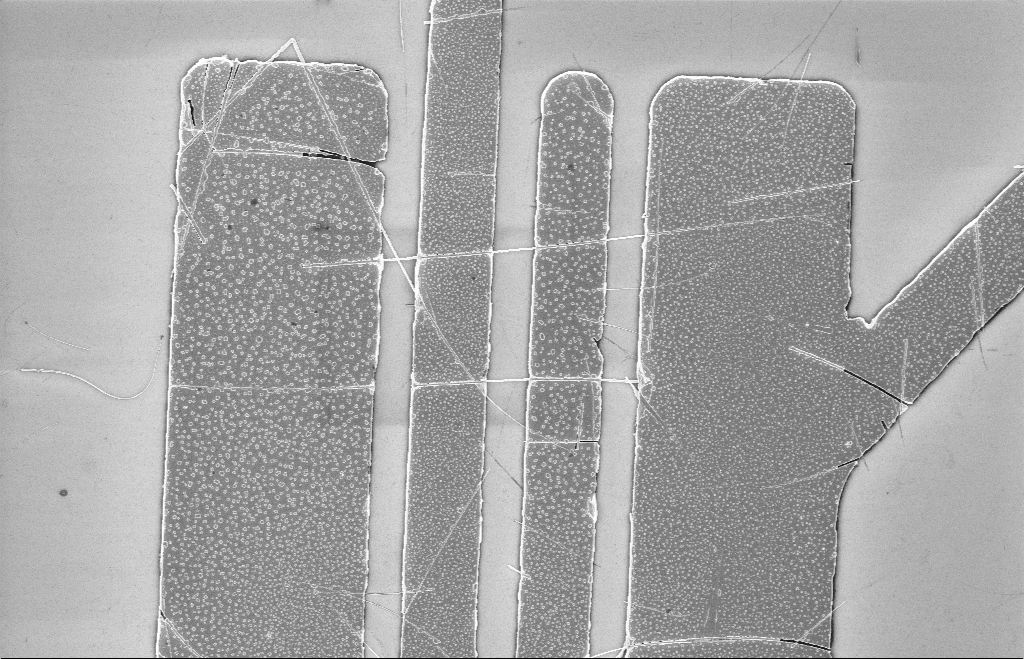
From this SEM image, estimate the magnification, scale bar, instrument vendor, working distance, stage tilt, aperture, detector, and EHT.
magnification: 6.94 K X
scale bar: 2000 nm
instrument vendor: Zeiss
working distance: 8 mm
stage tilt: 0°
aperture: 20 µm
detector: InLens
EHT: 5 kV